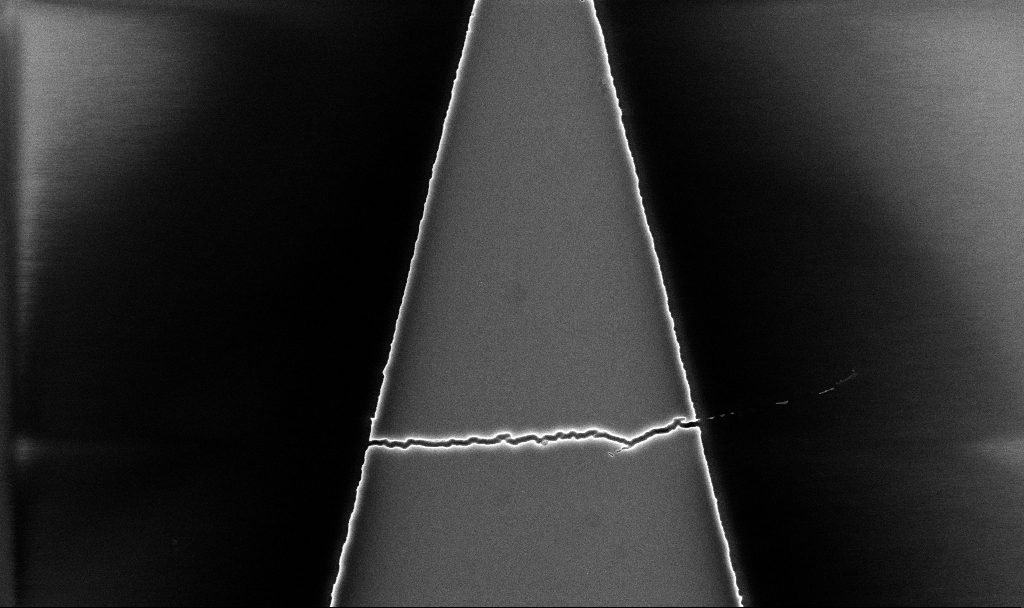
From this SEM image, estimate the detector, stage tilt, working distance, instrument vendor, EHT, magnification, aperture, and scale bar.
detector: InLens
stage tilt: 0°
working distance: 5.2 mm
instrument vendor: Zeiss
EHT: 5 kV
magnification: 13.08 K X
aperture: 30 µm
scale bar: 2000 nm